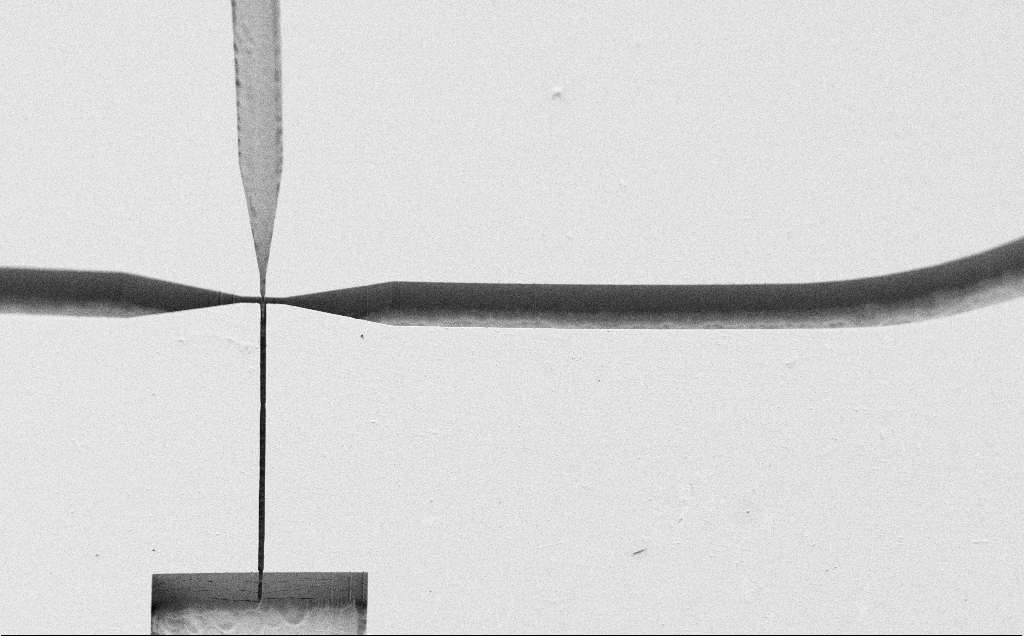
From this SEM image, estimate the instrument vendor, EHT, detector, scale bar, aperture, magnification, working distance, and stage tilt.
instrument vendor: Zeiss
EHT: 1.2 kV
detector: SE2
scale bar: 200000 nm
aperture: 30 µm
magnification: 0.191 K X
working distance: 5 mm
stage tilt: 45°